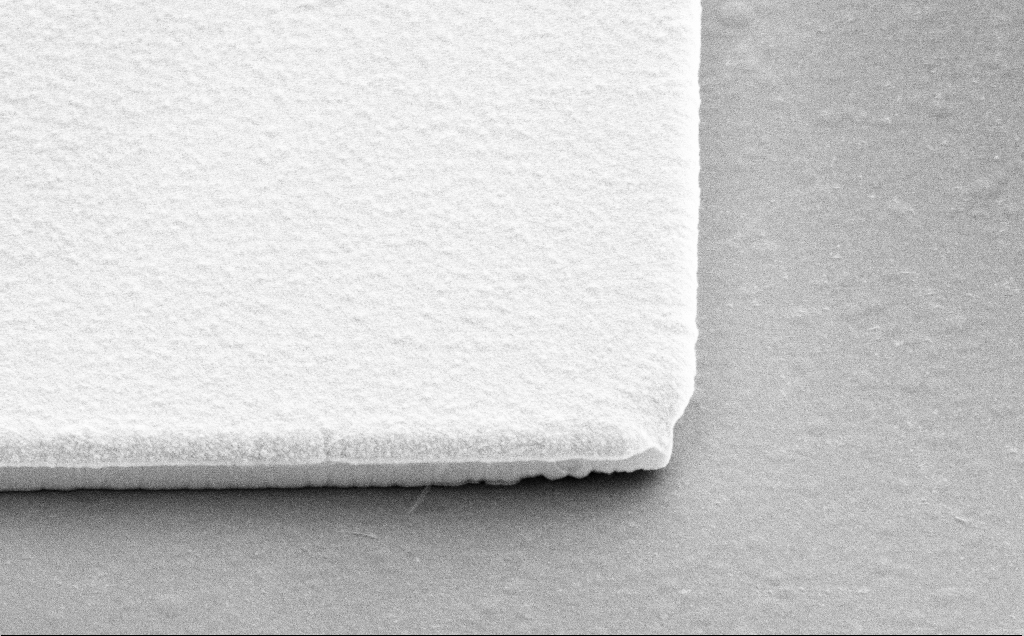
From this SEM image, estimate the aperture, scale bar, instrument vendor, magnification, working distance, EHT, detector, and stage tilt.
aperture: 30 µm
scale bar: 2000 nm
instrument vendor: Zeiss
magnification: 13.29 K X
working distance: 11 mm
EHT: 10 kV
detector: SE2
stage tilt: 45°